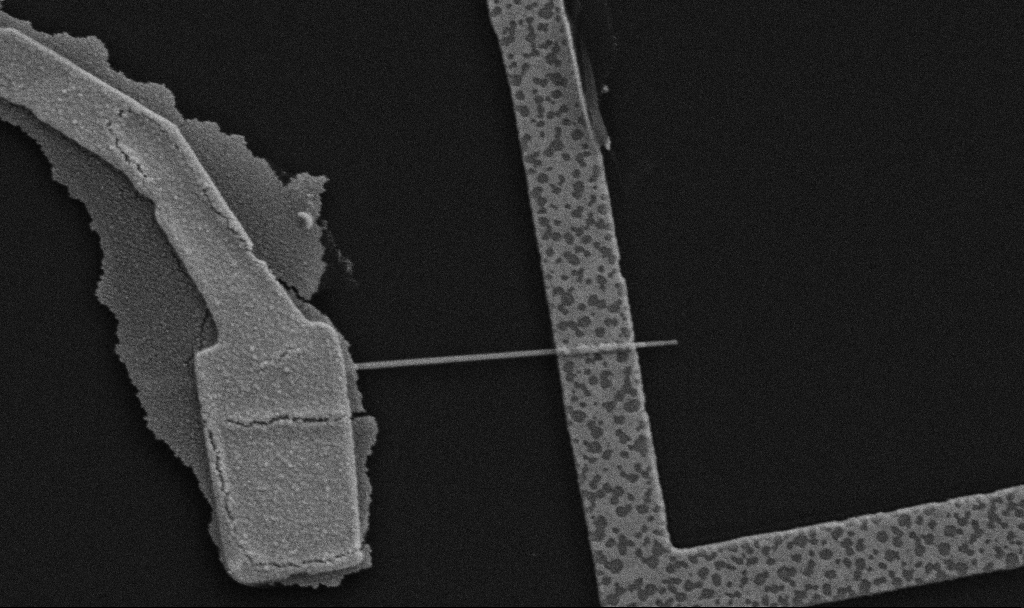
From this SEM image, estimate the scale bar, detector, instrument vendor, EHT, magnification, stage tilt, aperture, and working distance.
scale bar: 1000 nm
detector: SE2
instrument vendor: Zeiss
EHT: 5 kV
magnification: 30 K X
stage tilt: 0°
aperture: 30 µm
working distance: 10.7 mm